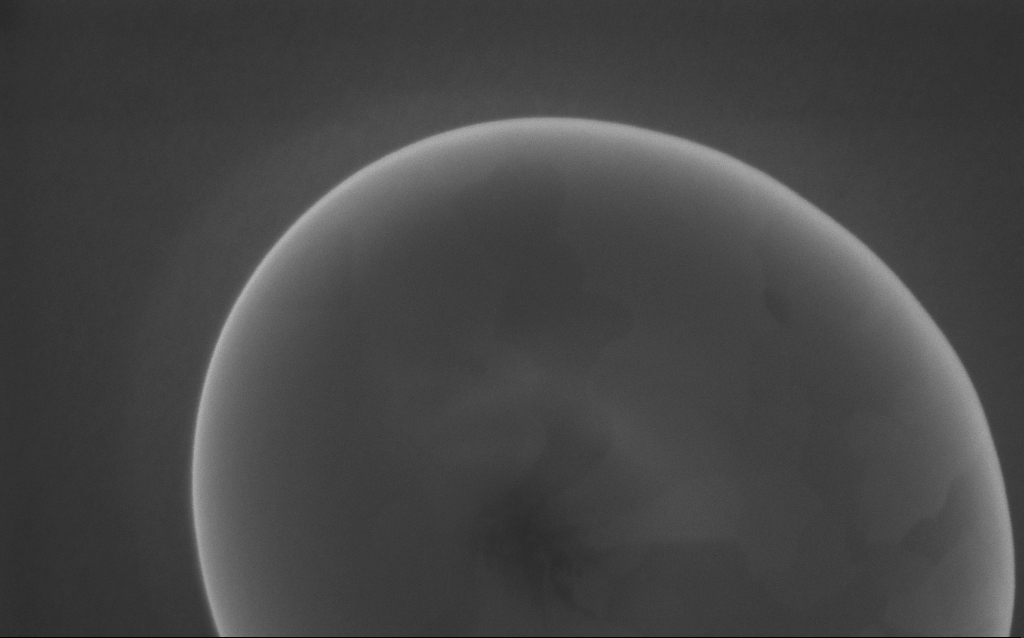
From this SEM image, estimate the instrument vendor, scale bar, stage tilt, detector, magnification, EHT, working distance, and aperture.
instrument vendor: Zeiss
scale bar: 200 nm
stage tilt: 0°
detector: InLens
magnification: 180 K X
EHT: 5 kV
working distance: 3 mm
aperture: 30 µm